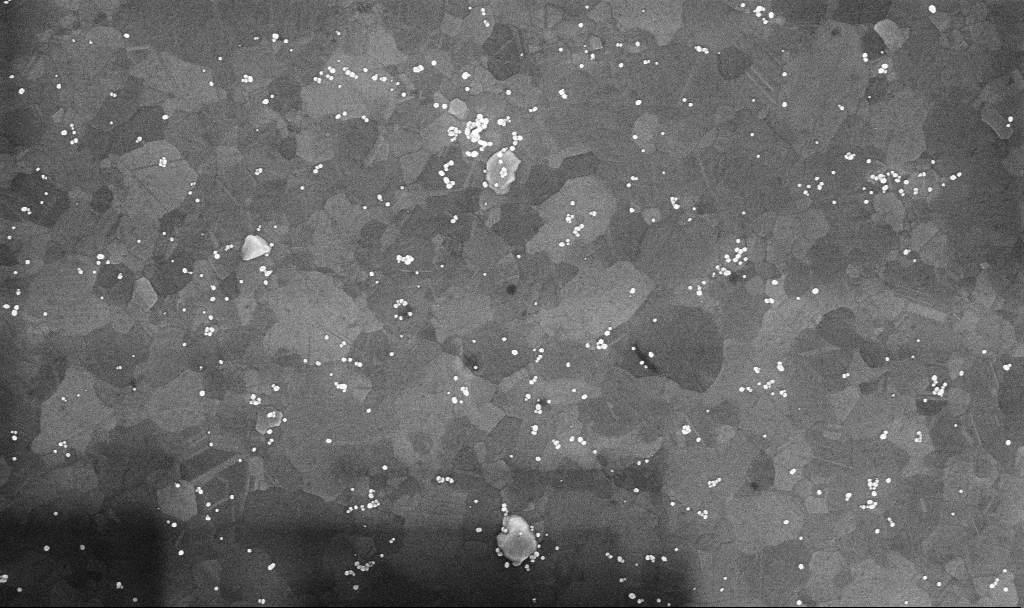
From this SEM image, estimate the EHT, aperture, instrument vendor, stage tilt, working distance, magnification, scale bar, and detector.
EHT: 10 kV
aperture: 30 µm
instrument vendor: Zeiss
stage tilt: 0°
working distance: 3.4 mm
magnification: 70.63 K X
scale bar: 1000 nm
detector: InLens